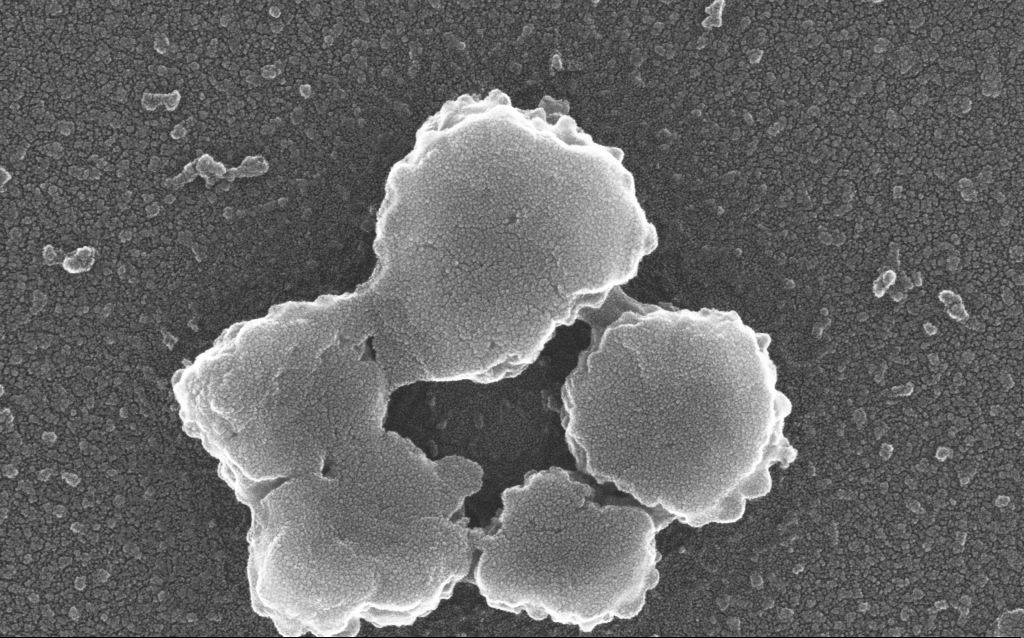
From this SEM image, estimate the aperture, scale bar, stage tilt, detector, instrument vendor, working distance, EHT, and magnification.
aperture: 30 µm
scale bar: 200 nm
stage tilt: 0°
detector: InLens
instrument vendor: Zeiss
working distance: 1.6 mm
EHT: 20 kV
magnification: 200 K X